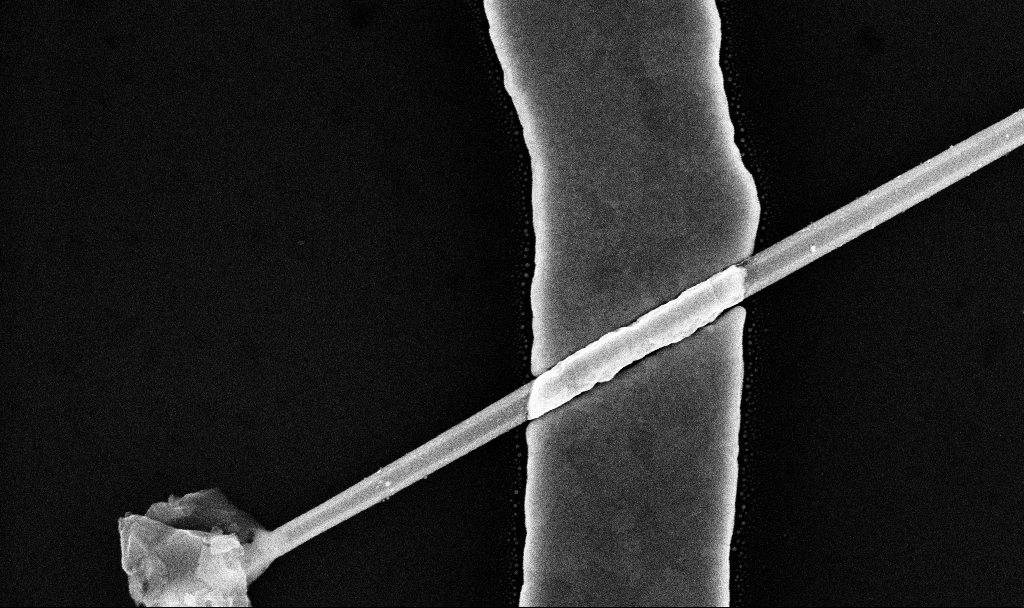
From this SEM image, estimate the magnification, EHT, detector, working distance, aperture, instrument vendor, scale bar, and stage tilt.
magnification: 89.4 K X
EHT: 10 kV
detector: InLens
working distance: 7.7 mm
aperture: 30 µm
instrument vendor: Zeiss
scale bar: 200 nm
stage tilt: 0°